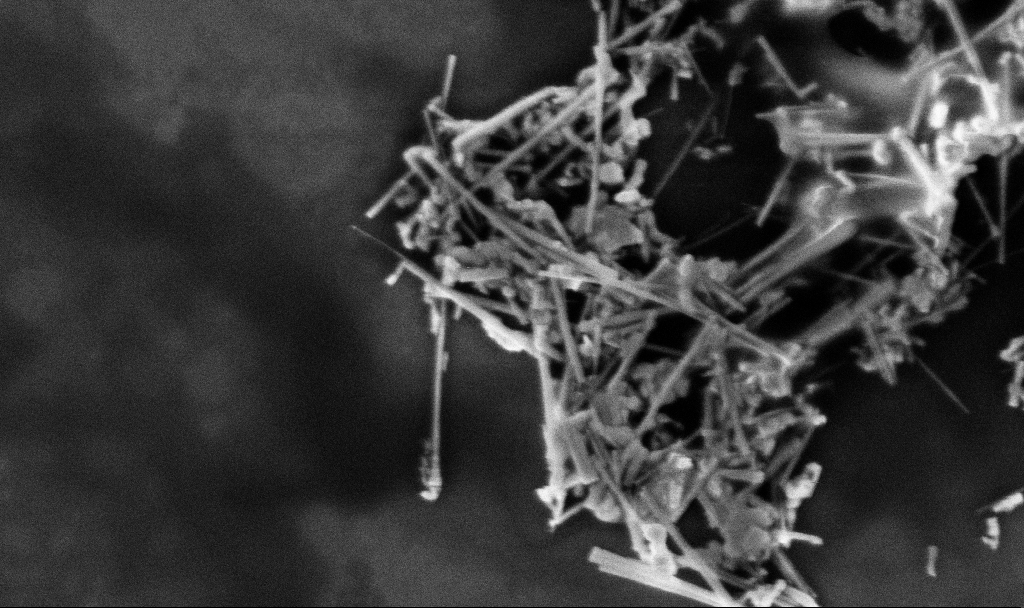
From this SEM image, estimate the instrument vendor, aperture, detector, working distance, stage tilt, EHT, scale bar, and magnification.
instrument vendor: Zeiss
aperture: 30 µm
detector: InLens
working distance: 3.3 mm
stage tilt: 0°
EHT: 3 kV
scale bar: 1000 nm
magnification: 64.77 K X